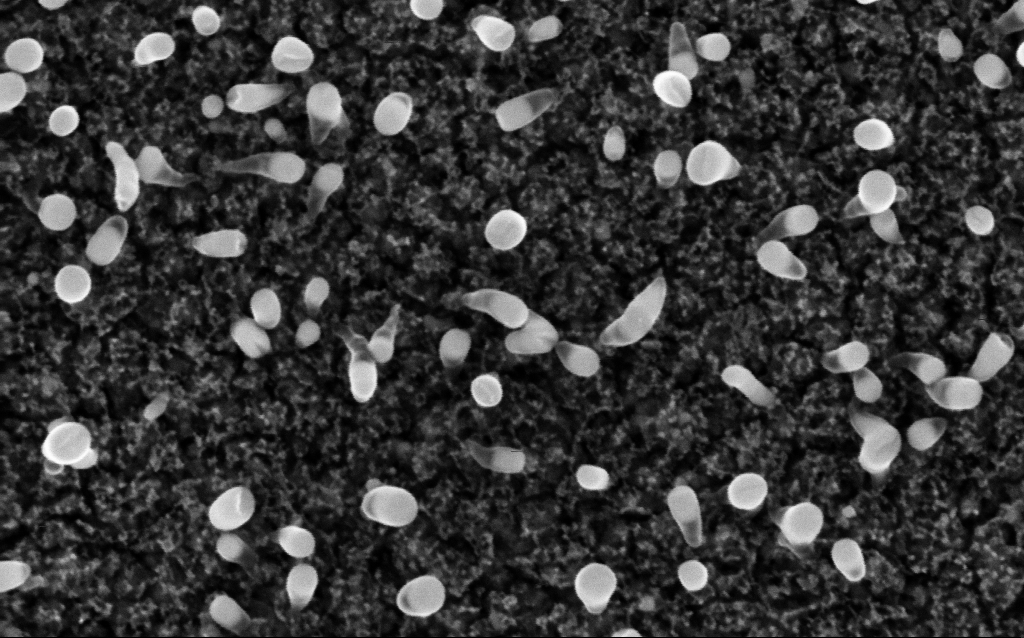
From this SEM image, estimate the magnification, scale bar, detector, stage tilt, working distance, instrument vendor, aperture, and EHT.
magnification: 200 K X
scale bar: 100 nm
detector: InLens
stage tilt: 0°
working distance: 1.9 mm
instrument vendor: Zeiss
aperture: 30 µm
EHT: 5 kV